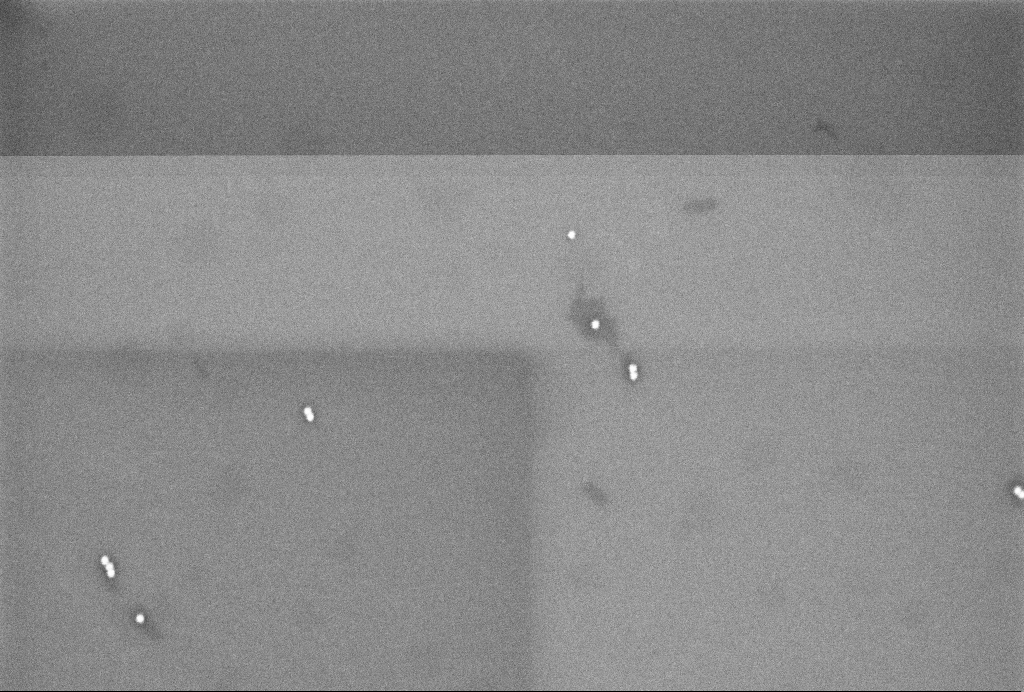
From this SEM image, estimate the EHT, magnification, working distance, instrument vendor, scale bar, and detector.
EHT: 3 kV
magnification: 102.77 K X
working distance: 3.2 mm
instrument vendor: Zeiss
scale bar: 200 nm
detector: InLens